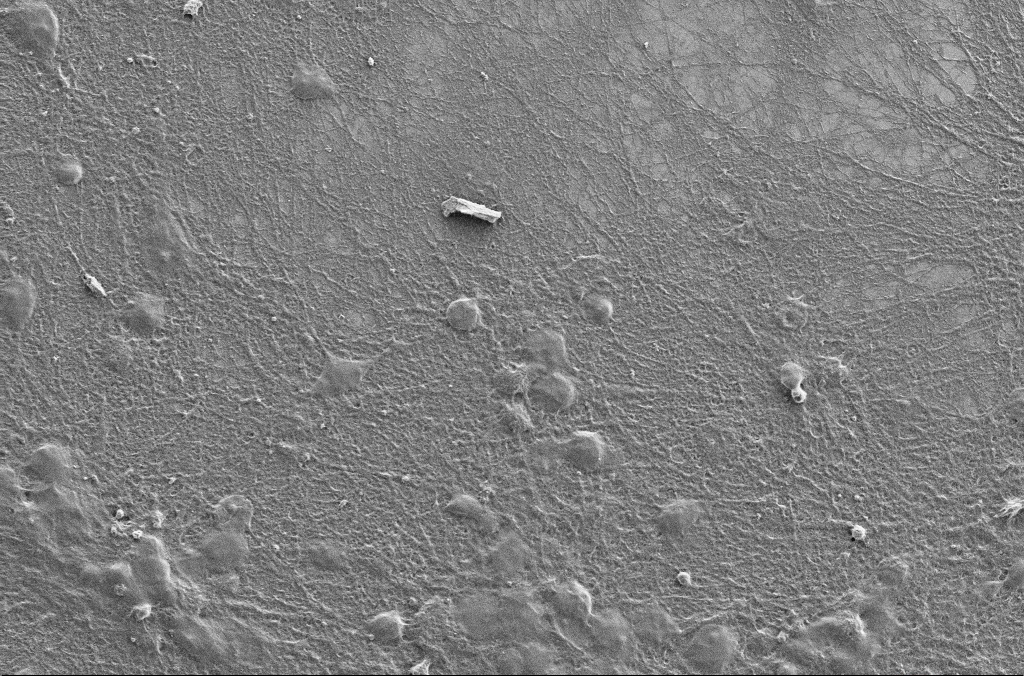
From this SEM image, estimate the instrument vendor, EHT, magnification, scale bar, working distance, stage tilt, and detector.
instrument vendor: Zeiss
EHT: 3 kV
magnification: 1 K X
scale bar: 20000 nm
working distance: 3 mm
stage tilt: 0°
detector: SE2